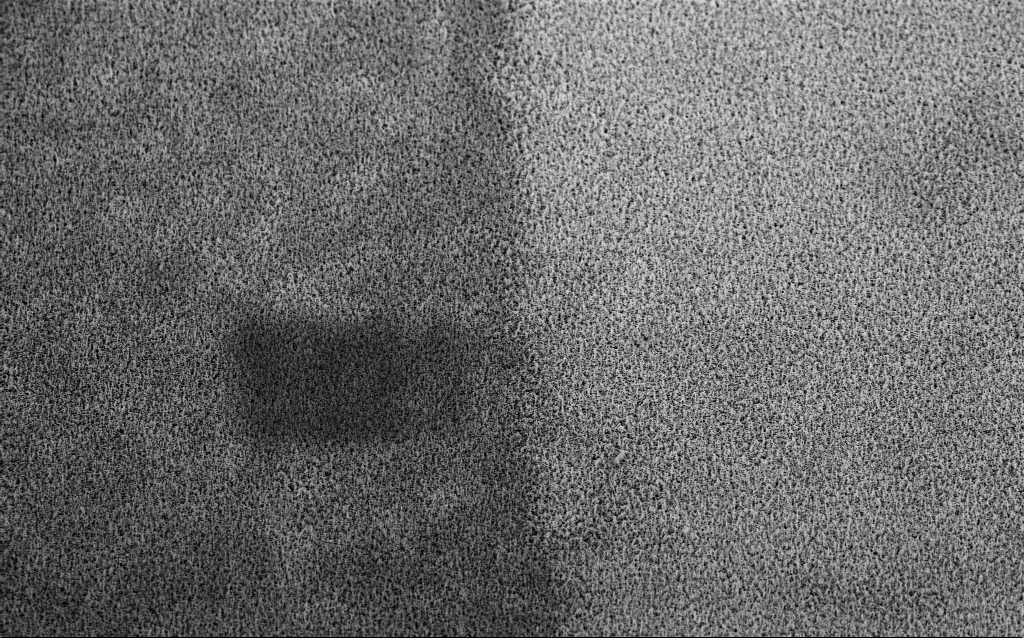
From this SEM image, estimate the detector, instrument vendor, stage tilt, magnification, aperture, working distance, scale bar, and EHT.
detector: InLens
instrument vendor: Zeiss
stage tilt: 35°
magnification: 4.37 K X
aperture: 30 µm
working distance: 7.3 mm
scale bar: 10000 nm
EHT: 10 kV